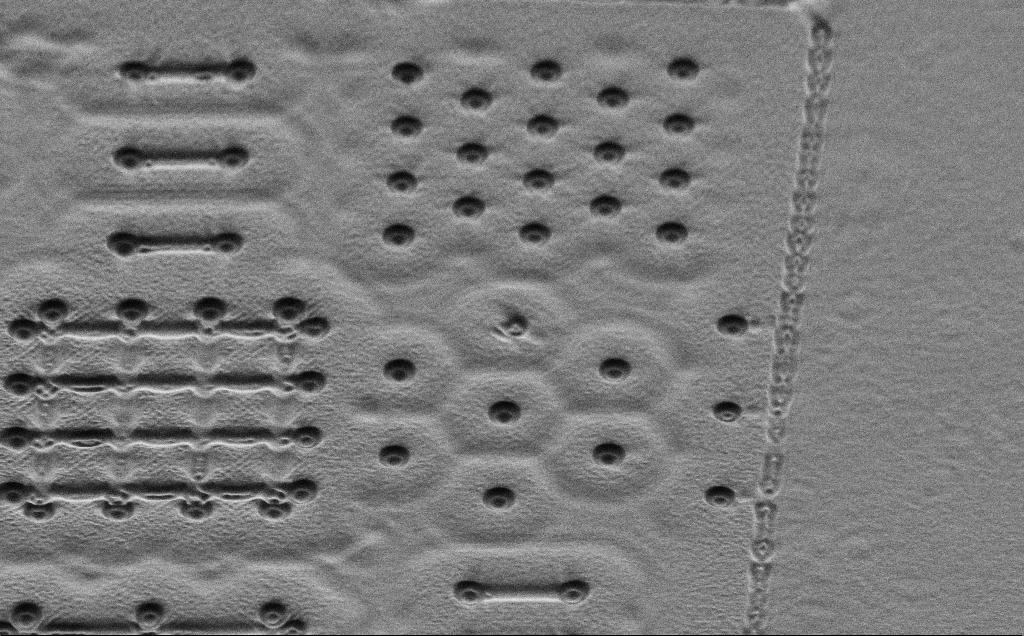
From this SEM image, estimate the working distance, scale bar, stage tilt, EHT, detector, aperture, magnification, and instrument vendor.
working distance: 6 mm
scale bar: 10000 nm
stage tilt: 45°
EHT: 1 kV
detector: SE2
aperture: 30 µm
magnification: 4.5 K X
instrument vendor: Zeiss